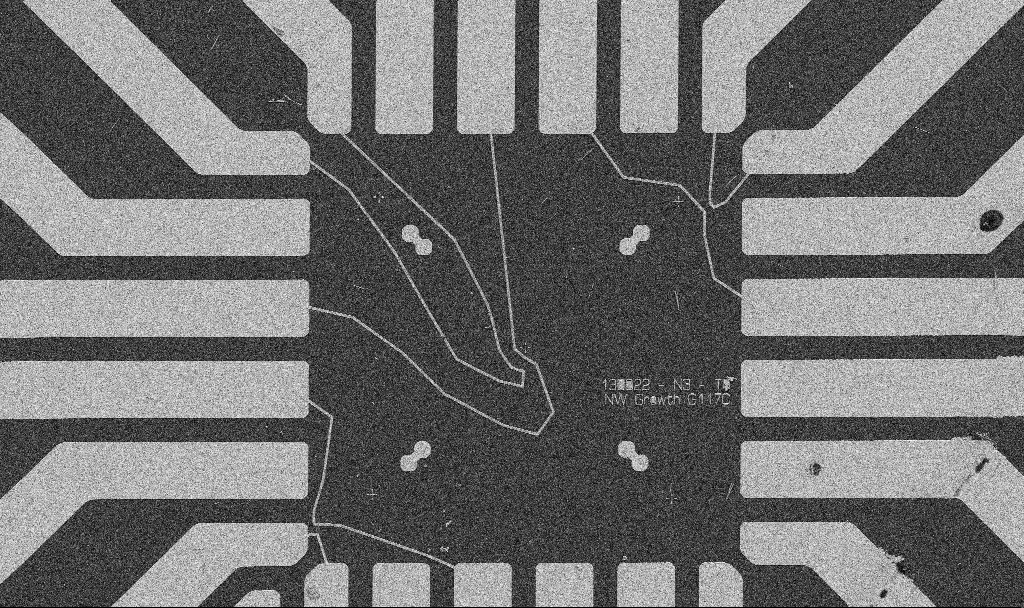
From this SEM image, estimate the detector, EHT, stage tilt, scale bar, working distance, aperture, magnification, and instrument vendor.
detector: SE2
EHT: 5 kV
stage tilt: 0°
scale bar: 20000 nm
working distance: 8.7 mm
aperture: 30 µm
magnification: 1 K X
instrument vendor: Zeiss